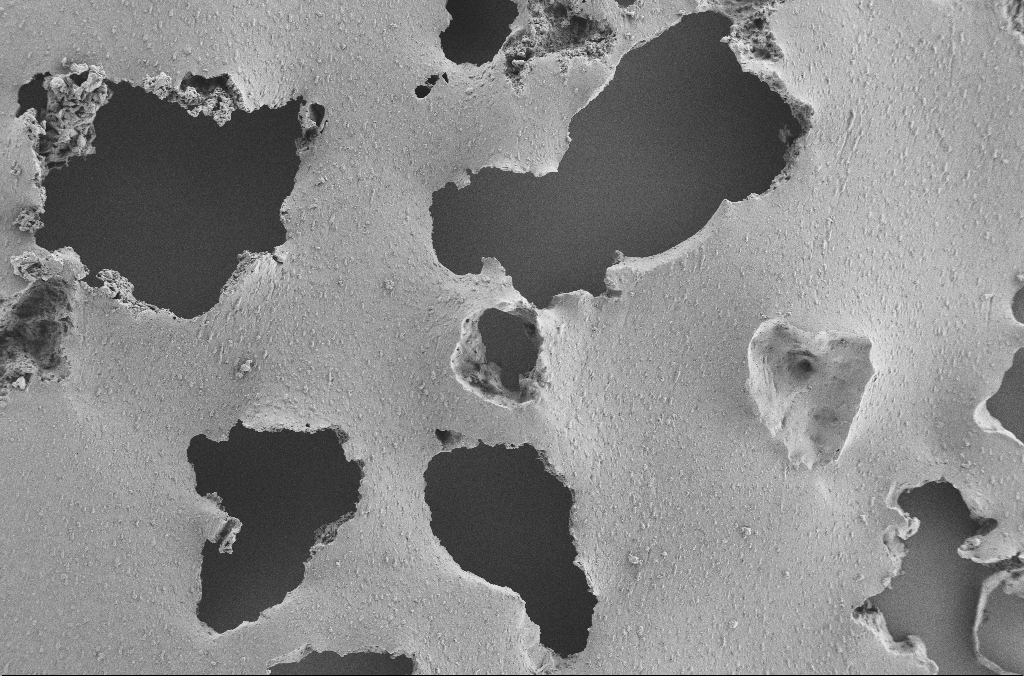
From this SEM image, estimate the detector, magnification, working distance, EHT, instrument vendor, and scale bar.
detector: SE2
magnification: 0.25 K X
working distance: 3.6 mm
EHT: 2 kV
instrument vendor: Zeiss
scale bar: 100000 nm